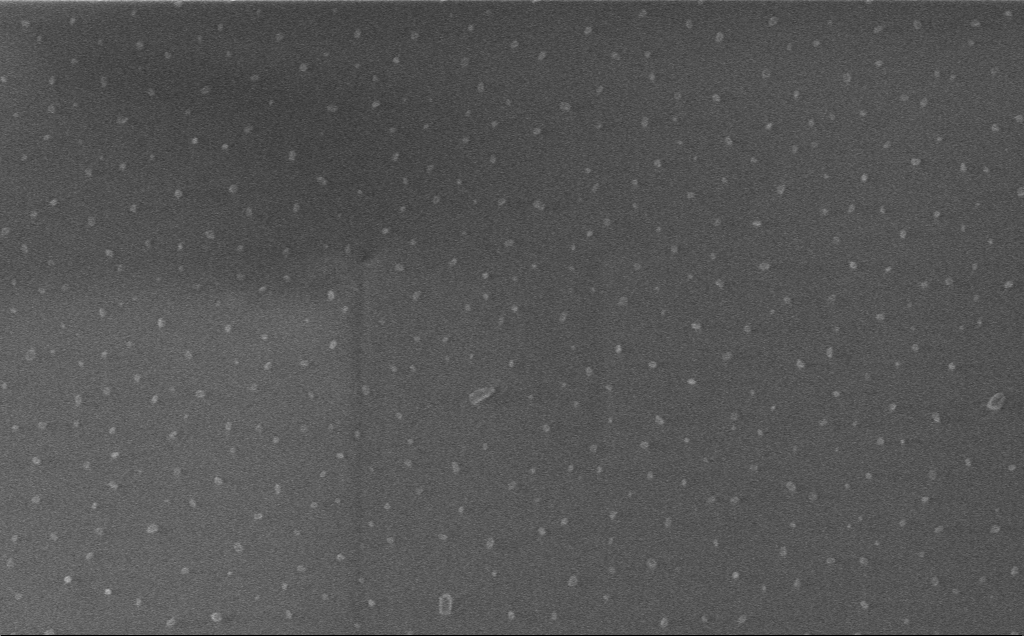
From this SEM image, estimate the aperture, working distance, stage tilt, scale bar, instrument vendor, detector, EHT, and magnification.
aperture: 30 µm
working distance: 3 mm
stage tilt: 0°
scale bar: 1000 nm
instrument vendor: Zeiss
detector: InLens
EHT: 1 kV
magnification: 37.69 K X